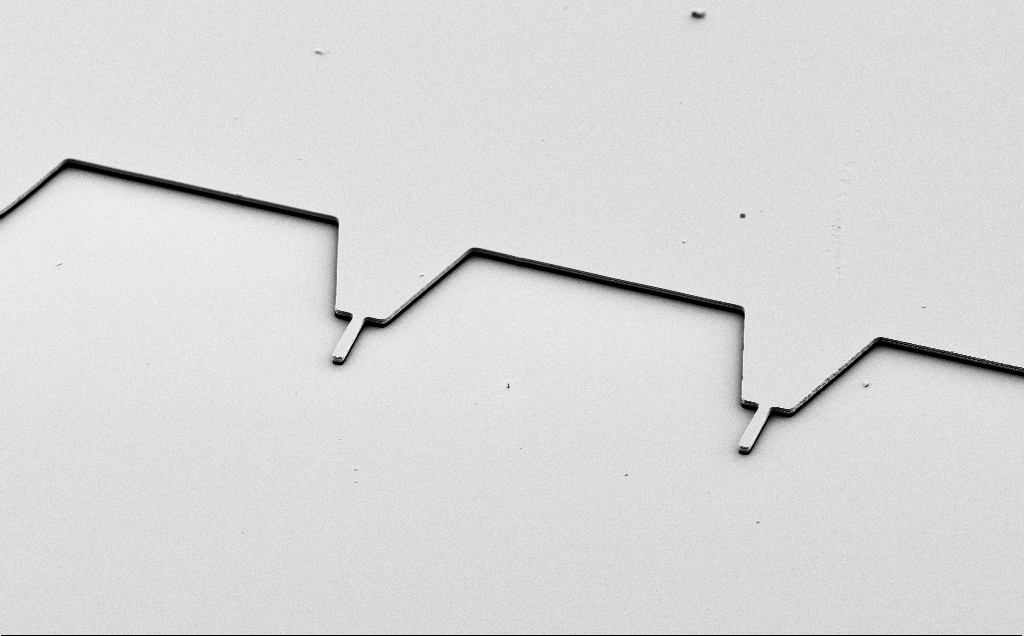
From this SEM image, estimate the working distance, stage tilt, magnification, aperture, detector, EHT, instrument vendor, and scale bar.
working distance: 10 mm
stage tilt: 50°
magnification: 0.99 K X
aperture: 30 µm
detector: SE2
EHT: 5 kV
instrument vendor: Zeiss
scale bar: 20000 nm